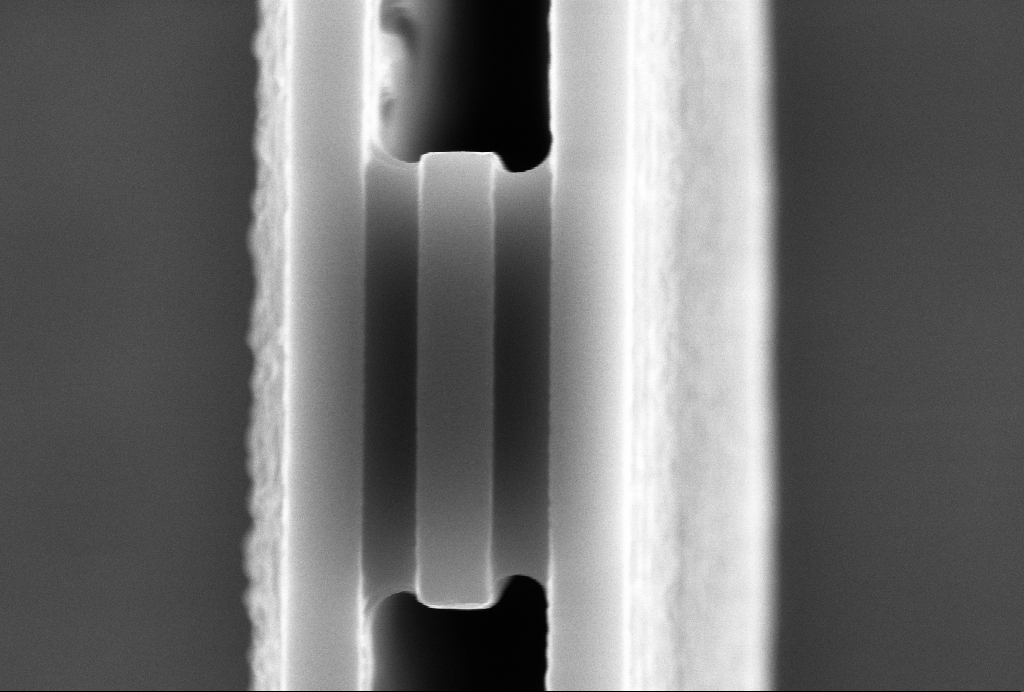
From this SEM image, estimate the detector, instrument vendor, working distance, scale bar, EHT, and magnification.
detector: InLens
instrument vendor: Zeiss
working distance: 5 mm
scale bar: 1000 nm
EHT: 10 kV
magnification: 55.74 K X